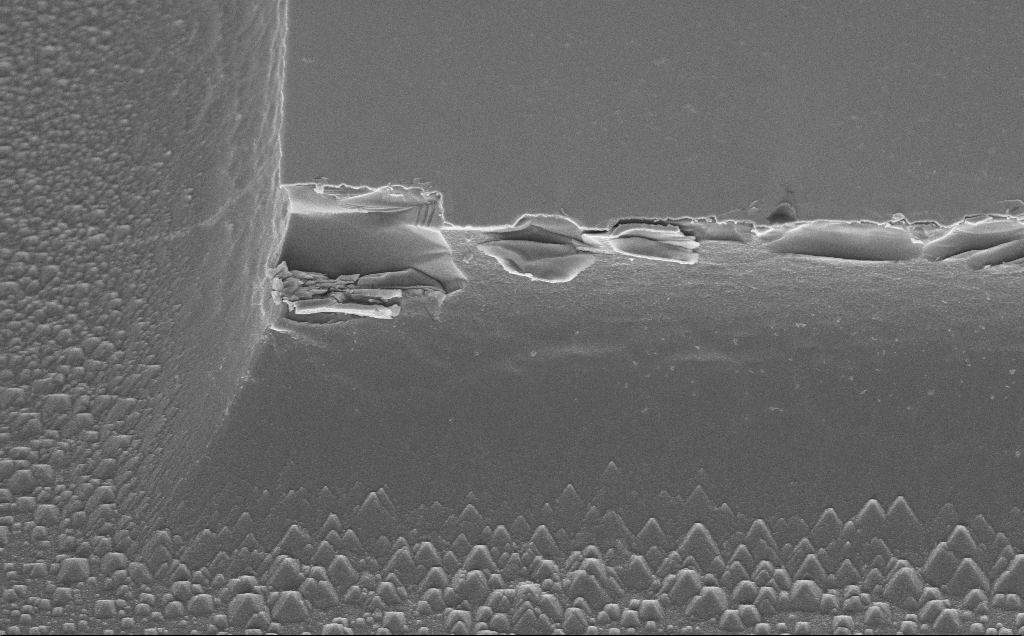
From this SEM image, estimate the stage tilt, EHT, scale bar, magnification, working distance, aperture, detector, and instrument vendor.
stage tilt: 45°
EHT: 5 kV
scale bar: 2000 nm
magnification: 8.22 K X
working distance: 13 mm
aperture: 30 µm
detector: InLens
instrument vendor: Zeiss